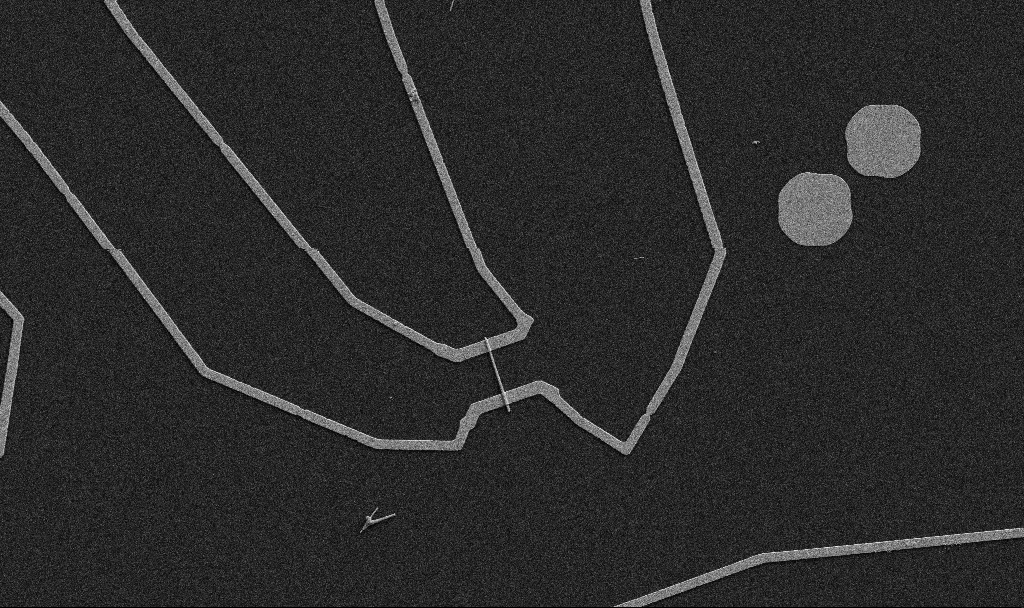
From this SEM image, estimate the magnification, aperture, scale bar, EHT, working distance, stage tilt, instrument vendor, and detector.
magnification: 5 K X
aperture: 30 µm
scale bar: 10000 nm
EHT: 5 kV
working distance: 10.7 mm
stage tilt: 0°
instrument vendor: Zeiss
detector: SE2